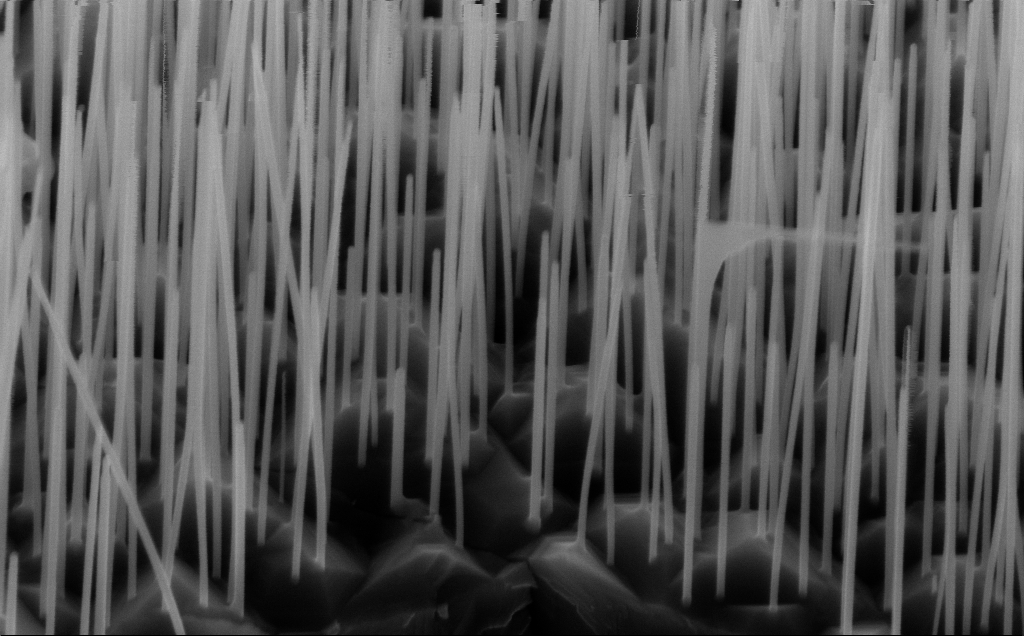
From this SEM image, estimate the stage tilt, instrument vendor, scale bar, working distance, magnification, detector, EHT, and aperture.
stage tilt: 45°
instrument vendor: Zeiss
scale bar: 1000 nm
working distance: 5 mm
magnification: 70.86 K X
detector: InLens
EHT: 10 kV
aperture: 30 µm